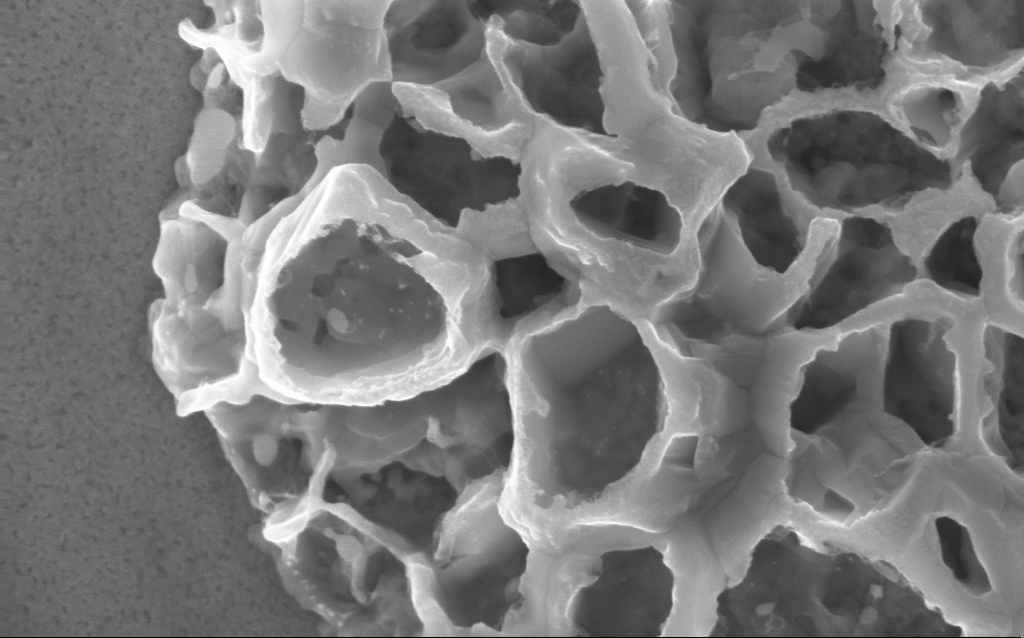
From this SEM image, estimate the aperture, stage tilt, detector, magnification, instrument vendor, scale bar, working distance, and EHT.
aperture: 30 µm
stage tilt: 0°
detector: InLens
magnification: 170.76 K X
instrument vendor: Zeiss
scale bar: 200 nm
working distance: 2 mm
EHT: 10 kV